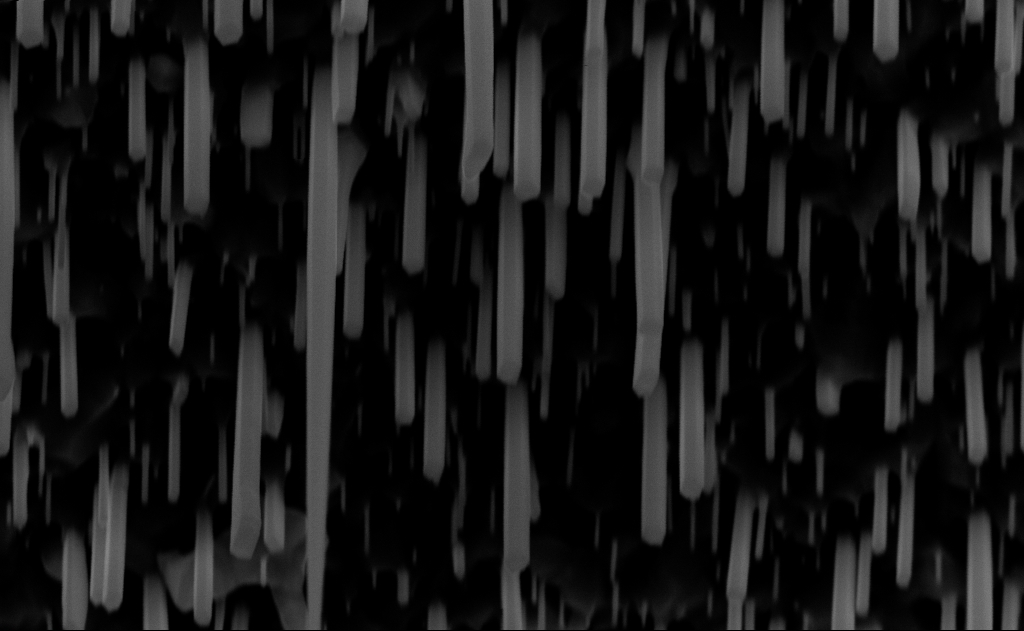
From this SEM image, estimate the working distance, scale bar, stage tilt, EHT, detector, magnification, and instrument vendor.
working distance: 9 mm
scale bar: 1000 nm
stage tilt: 0°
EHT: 10 kV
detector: InLens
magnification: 60 K X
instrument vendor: Zeiss